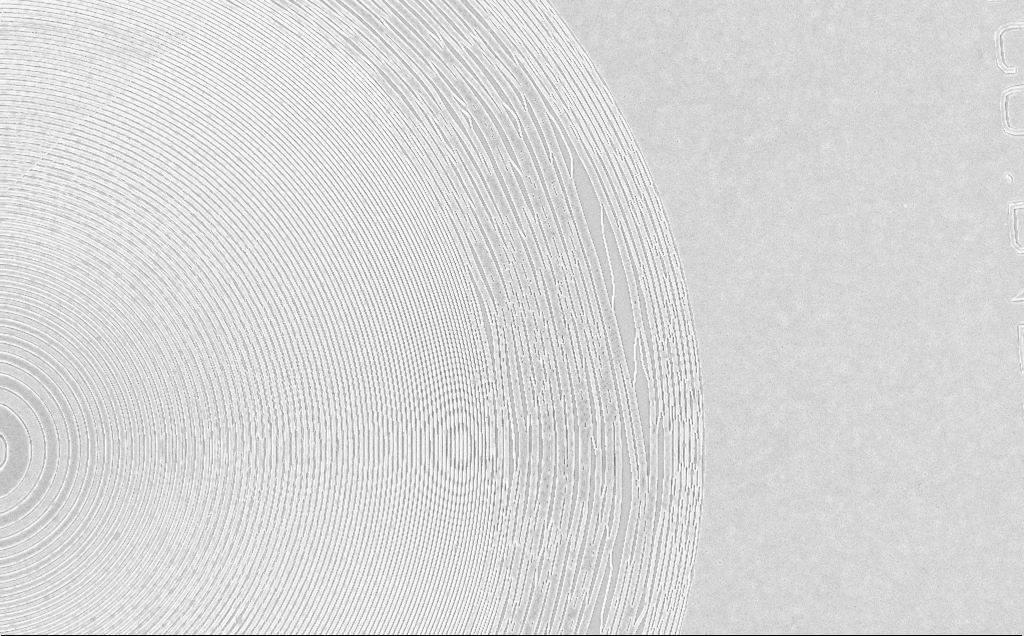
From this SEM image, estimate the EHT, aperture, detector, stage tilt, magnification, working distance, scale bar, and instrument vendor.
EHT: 5 kV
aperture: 30 µm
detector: InLens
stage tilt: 0°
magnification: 3.32 K X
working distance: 6 mm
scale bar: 10000 nm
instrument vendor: Zeiss